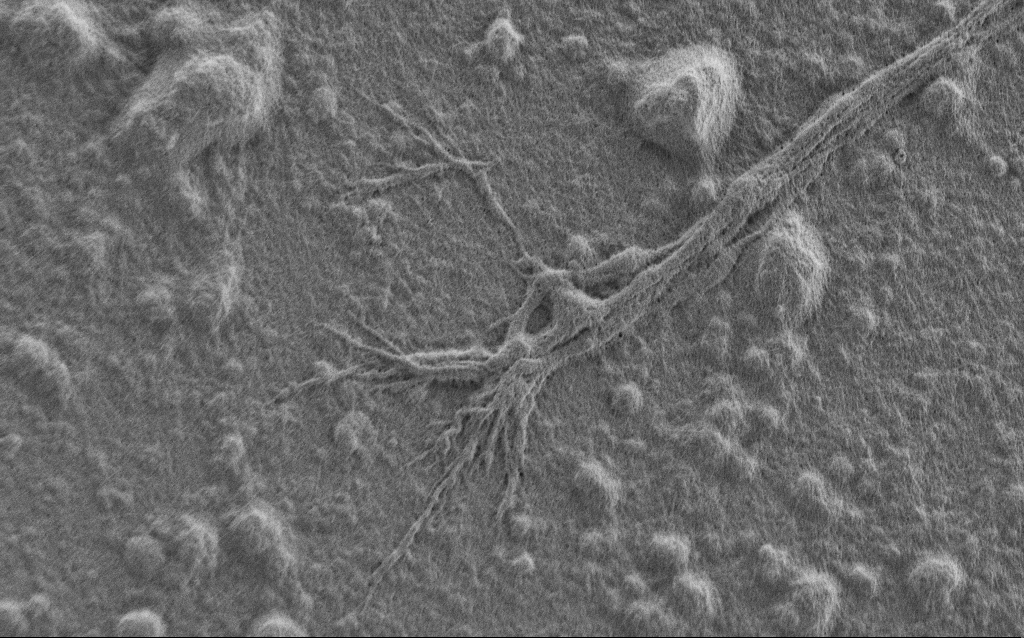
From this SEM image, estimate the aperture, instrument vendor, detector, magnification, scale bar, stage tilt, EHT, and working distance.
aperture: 30 µm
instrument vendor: Zeiss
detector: SE2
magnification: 7.5 K X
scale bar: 2000 nm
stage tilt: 0°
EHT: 0.9 kV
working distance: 7 mm